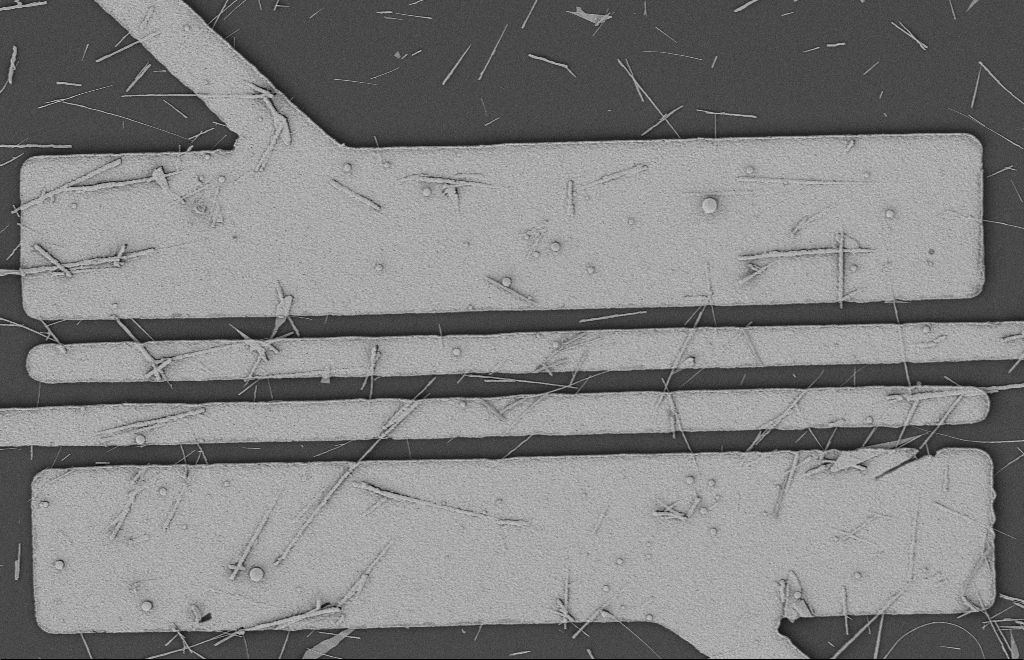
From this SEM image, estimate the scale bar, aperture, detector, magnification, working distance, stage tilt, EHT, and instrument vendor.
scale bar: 2000 nm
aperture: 20 µm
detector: SE2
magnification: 5.73 K X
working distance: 12 mm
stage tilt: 0°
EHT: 2 kV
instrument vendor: Zeiss